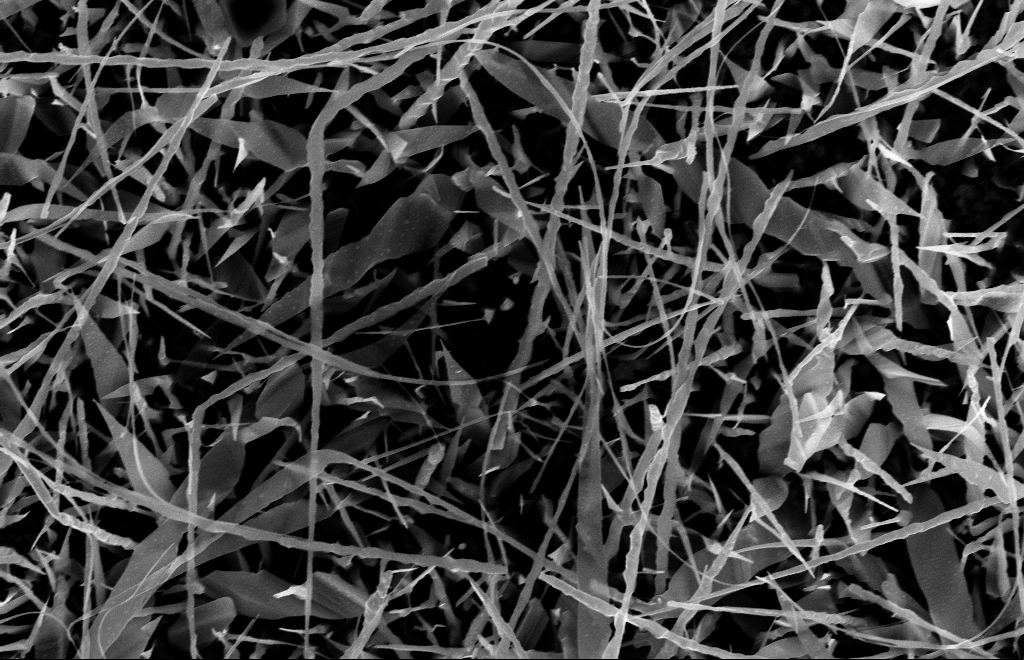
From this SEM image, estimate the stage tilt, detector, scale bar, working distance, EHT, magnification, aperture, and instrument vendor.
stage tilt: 0°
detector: InLens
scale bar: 2000 nm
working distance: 15 mm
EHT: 10 kV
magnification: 20 K X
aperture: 30 µm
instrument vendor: Zeiss